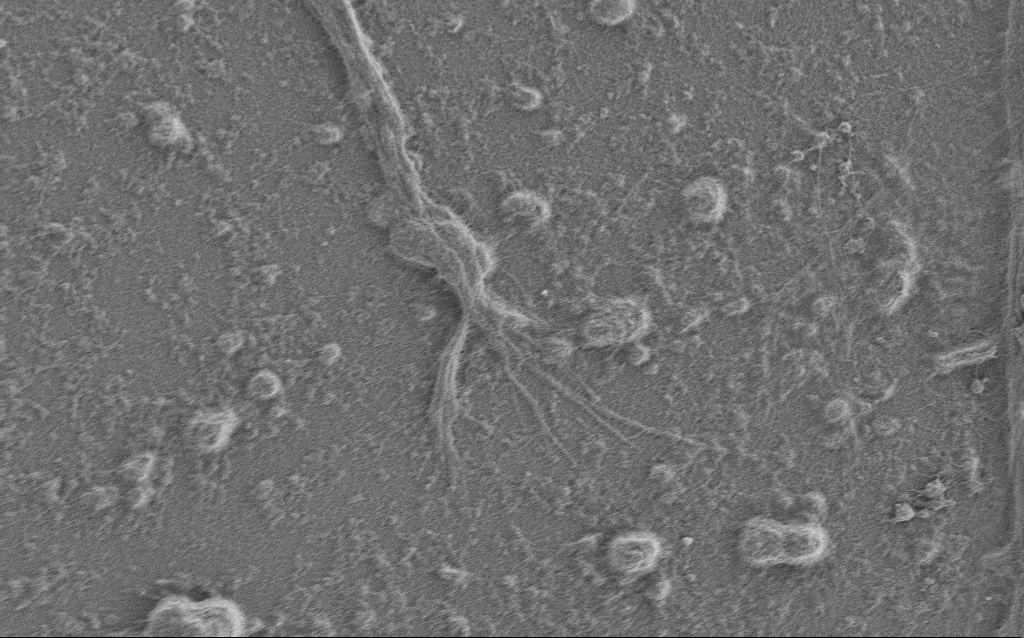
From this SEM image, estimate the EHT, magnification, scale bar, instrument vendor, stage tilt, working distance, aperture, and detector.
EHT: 1 kV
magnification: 7.5 K X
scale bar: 2000 nm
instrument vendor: Zeiss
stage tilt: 0°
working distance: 6 mm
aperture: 30 µm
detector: SE2